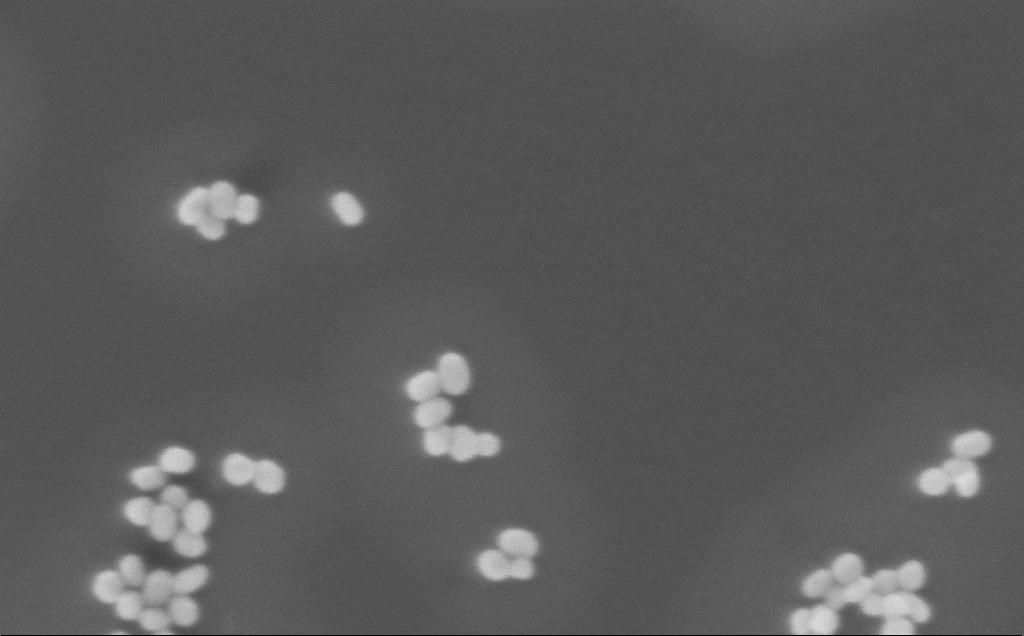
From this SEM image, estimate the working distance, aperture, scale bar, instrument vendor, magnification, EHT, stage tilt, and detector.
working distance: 5 mm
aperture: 30 µm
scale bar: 100 nm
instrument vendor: Zeiss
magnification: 300 K X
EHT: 3 kV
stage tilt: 0°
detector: InLens